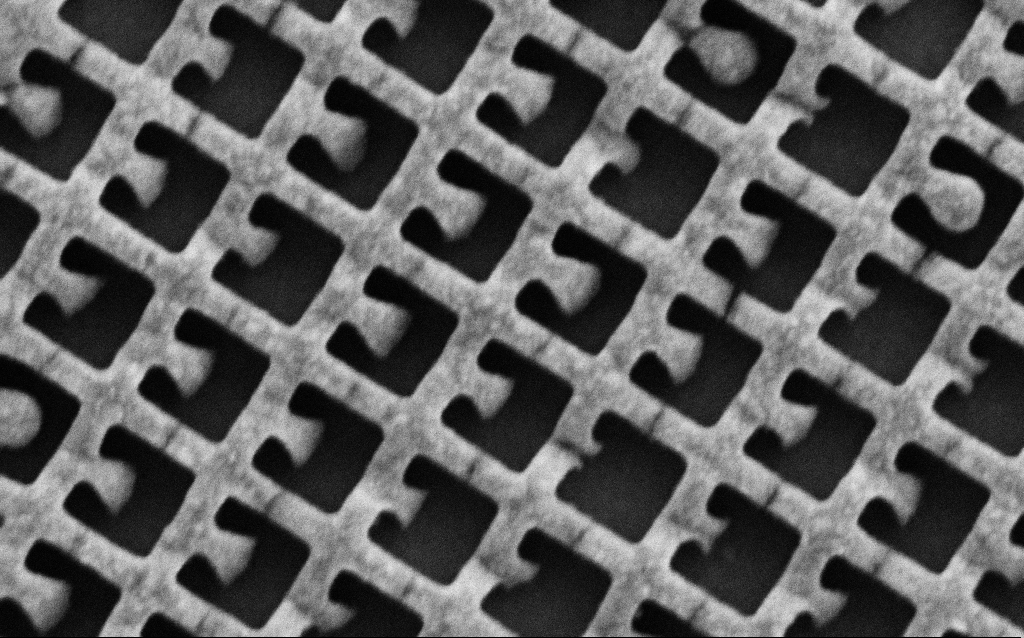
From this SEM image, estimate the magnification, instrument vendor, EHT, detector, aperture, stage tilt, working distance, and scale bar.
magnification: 108.85 K X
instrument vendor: Zeiss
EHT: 5 kV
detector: SE2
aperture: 30 µm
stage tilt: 0°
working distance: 6.1 mm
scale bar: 200 nm